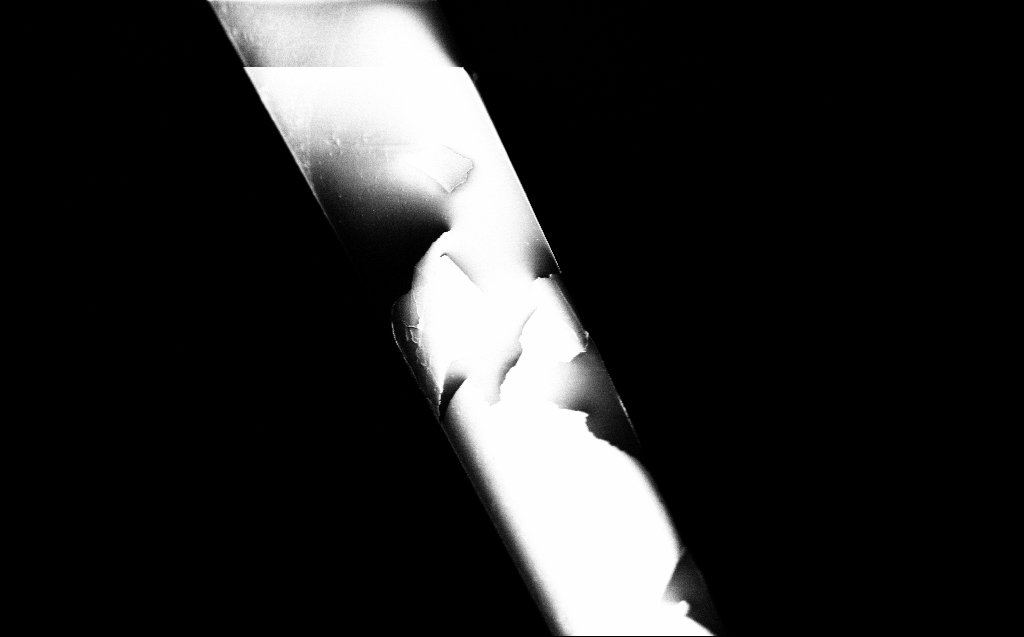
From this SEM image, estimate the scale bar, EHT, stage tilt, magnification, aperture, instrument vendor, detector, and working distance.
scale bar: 10000 nm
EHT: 0.8 kV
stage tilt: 45°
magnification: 2.5 K X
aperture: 30 µm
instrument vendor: Zeiss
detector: InLens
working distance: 3 mm